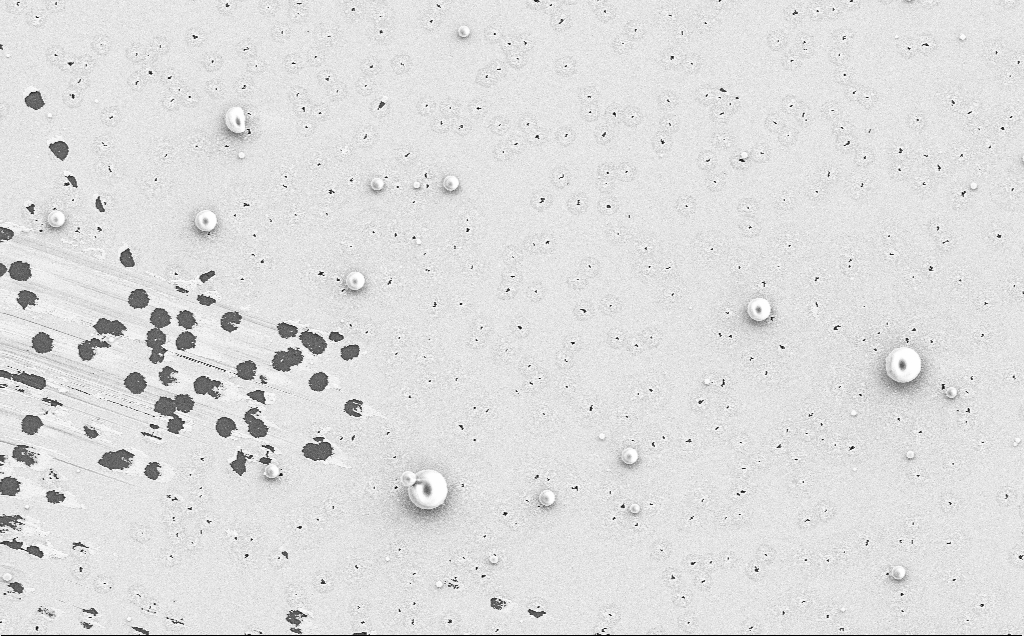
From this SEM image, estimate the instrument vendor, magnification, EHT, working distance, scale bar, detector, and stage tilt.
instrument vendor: Zeiss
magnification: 4.2 K X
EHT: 5 kV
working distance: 12 mm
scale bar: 10000 nm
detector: SE2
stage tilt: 0°